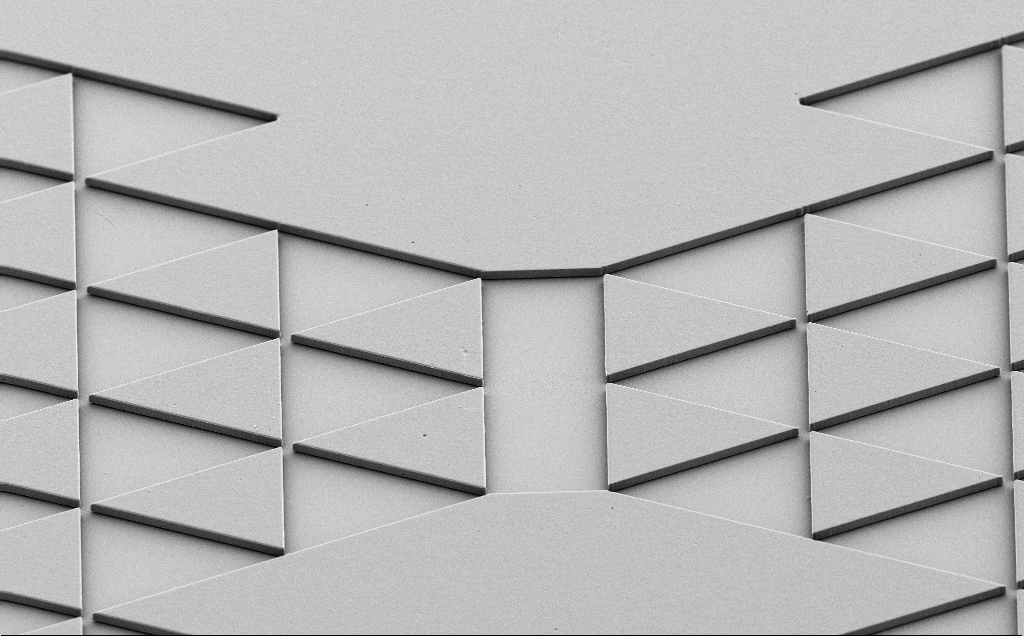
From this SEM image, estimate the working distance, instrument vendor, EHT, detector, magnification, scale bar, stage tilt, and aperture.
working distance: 9 mm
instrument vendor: Zeiss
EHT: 5 kV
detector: SE2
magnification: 0.883 K X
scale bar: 20000 nm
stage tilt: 40°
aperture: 30 µm